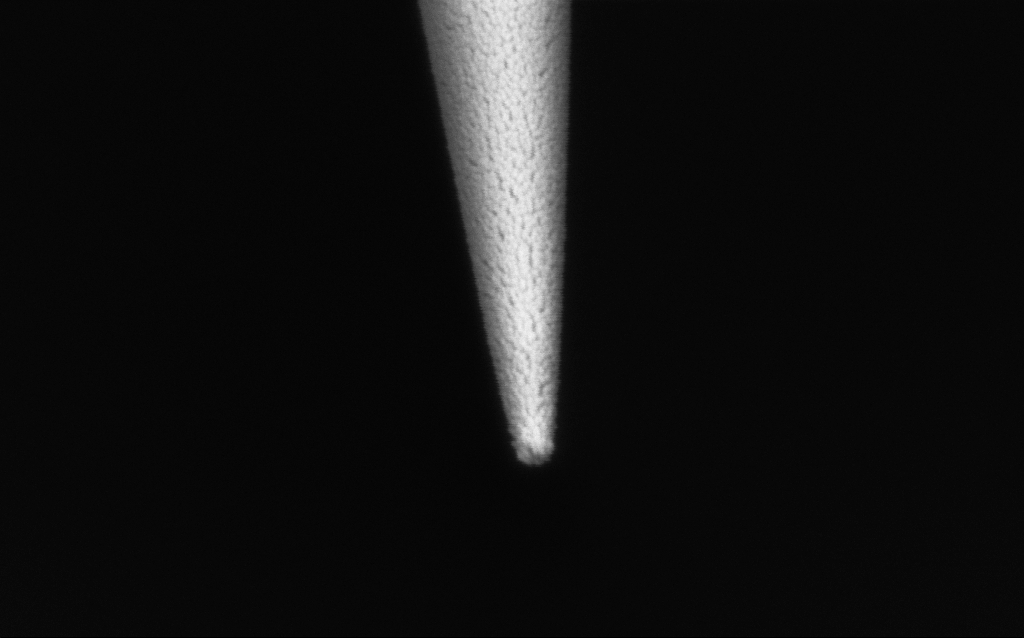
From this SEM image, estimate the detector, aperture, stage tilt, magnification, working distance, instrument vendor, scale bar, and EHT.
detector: InLens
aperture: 30 µm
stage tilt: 45°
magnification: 150 K X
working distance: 7.7 mm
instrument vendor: Zeiss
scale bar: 200 nm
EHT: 2 kV